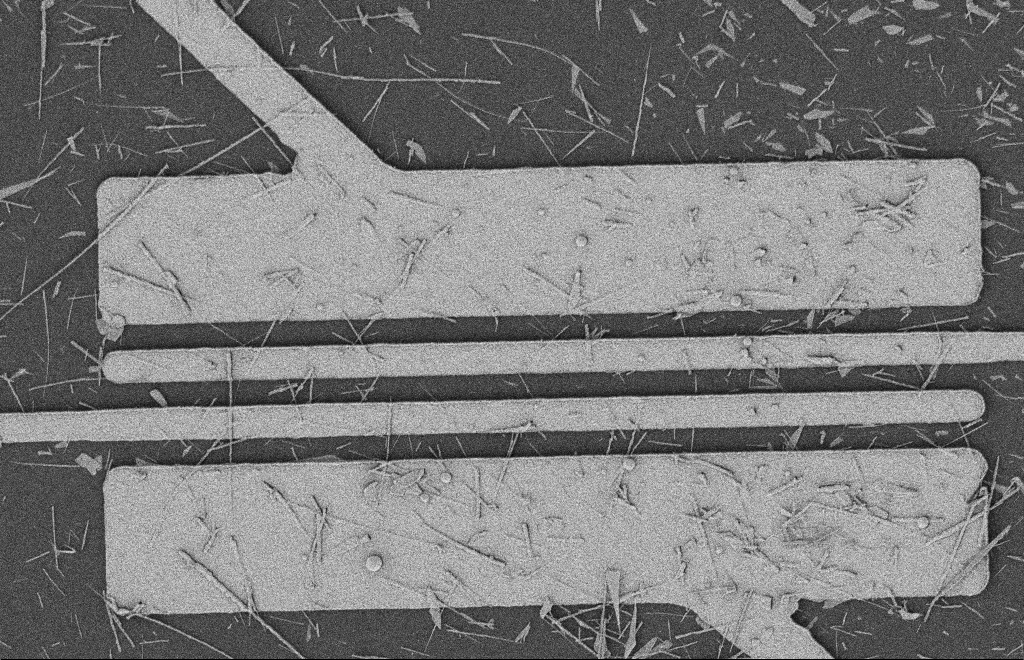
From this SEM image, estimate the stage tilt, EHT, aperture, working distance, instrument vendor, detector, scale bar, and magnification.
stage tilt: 0°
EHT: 2 kV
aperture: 20 µm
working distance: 12 mm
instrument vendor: Zeiss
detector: SE2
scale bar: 2000 nm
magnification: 5.29 K X